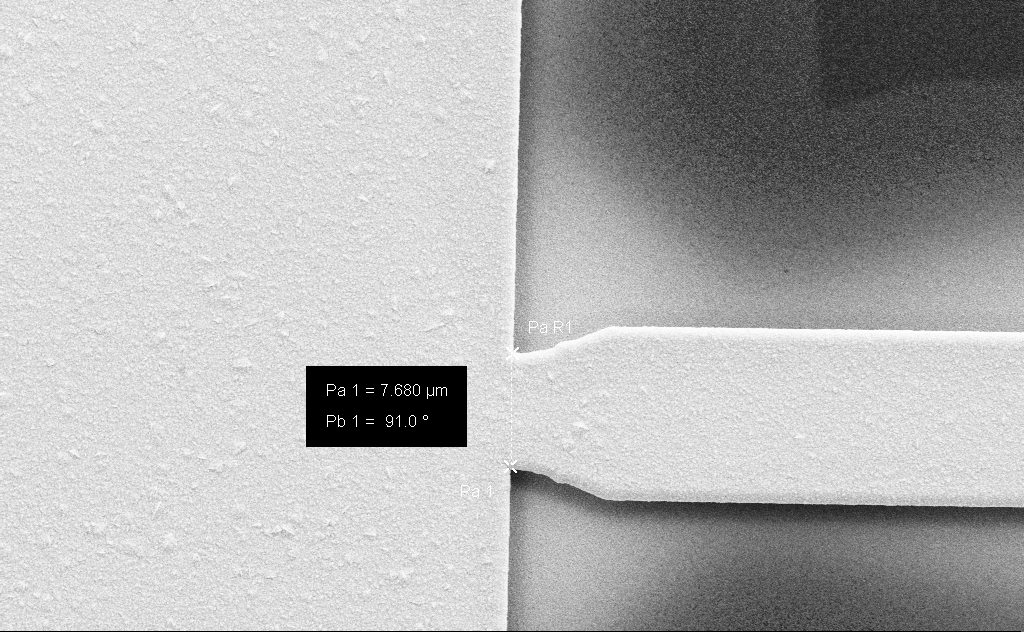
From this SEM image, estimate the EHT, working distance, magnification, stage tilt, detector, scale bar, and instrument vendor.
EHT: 10 kV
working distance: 11 mm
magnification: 5.4 K X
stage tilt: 0°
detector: SE2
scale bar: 10000 nm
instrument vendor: Zeiss